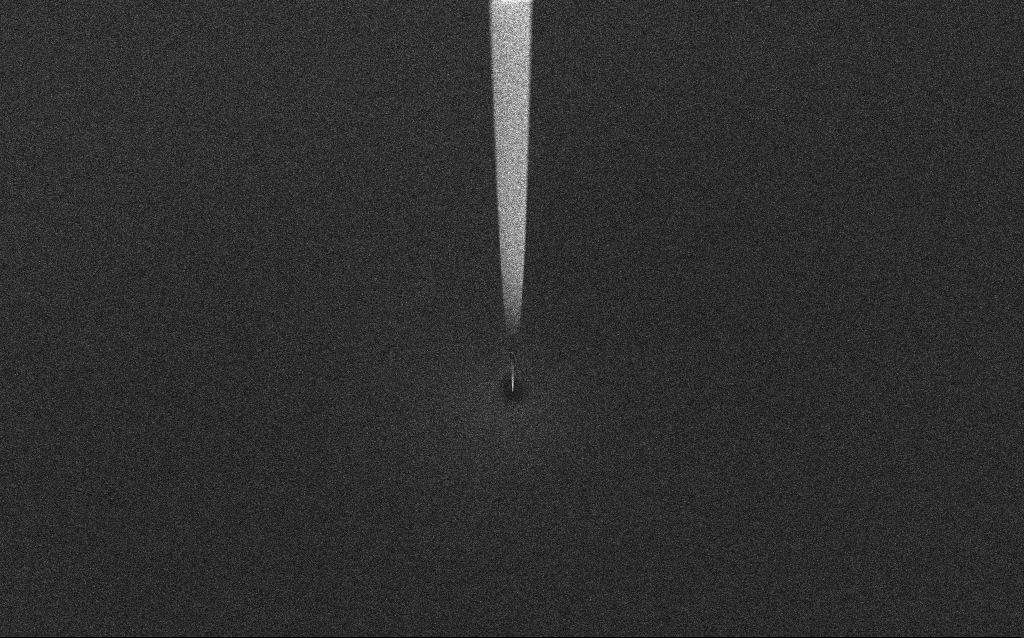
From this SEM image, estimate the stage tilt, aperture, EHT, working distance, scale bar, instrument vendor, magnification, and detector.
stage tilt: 45°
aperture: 30 µm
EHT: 1 kV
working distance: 6 mm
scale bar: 100000 nm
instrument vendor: Zeiss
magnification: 0.5 K X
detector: InLens